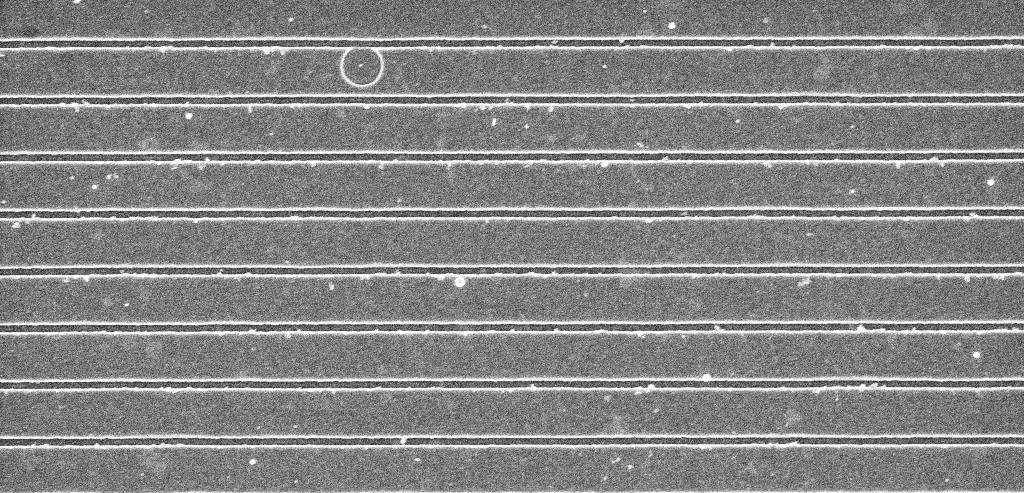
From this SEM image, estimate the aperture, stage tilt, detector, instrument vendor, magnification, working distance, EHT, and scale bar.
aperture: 30 µm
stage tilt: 0°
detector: InLens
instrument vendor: Zeiss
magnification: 26.16 K X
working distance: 5.3 mm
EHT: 5 kV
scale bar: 2000 nm